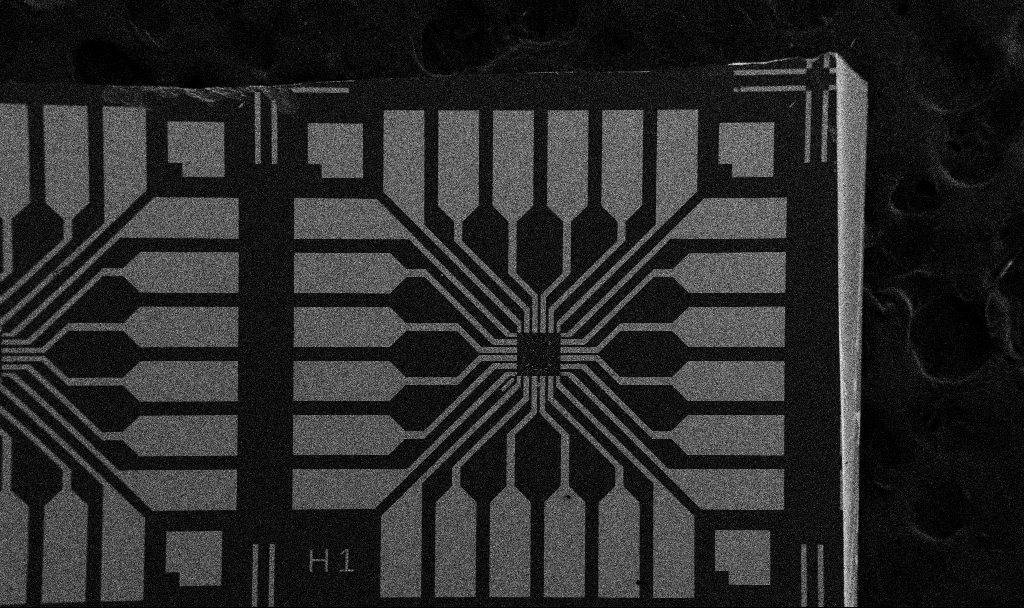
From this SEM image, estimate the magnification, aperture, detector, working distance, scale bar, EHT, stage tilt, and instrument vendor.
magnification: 0.1 K X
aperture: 30 µm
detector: SE2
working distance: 10.7 mm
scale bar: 200000 nm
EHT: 5 kV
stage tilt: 0°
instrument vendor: Zeiss